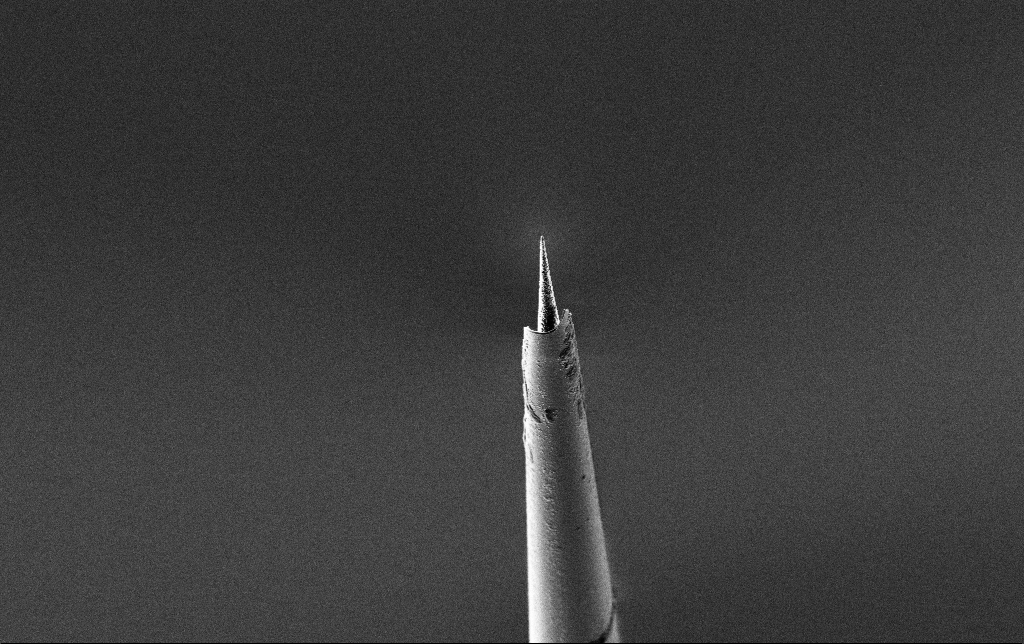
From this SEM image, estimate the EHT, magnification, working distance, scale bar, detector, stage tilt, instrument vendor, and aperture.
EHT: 2 kV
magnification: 5 K X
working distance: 7.4 mm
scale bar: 10000 nm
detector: SE2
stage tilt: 45°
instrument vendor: Zeiss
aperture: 30 µm